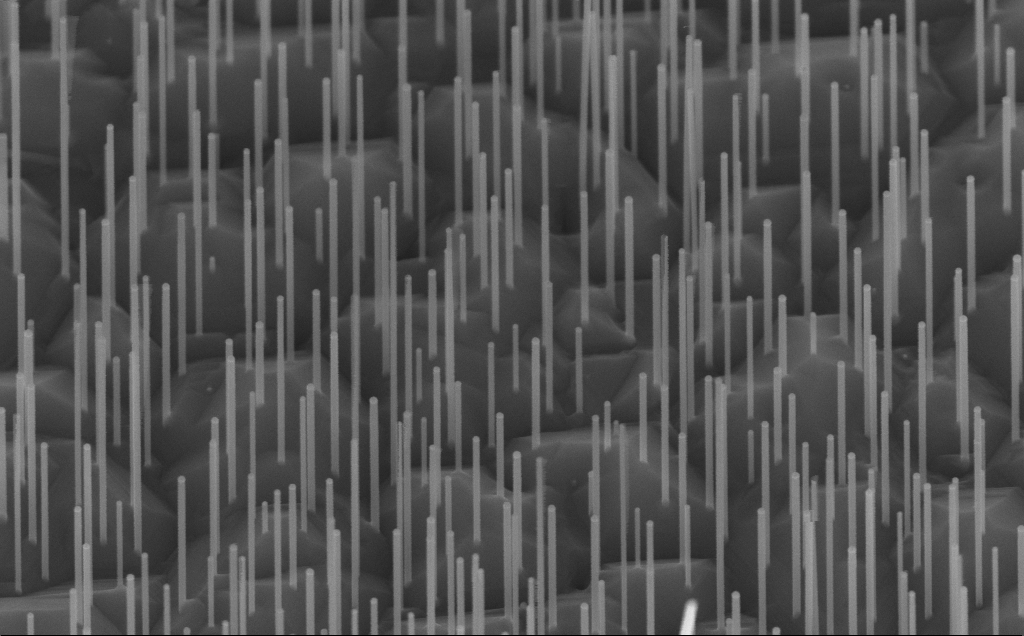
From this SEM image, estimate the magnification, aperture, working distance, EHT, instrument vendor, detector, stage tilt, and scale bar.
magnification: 79.85 K X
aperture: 30 µm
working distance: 8 mm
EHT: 10 kV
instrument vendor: Zeiss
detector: InLens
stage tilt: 45°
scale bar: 200 nm